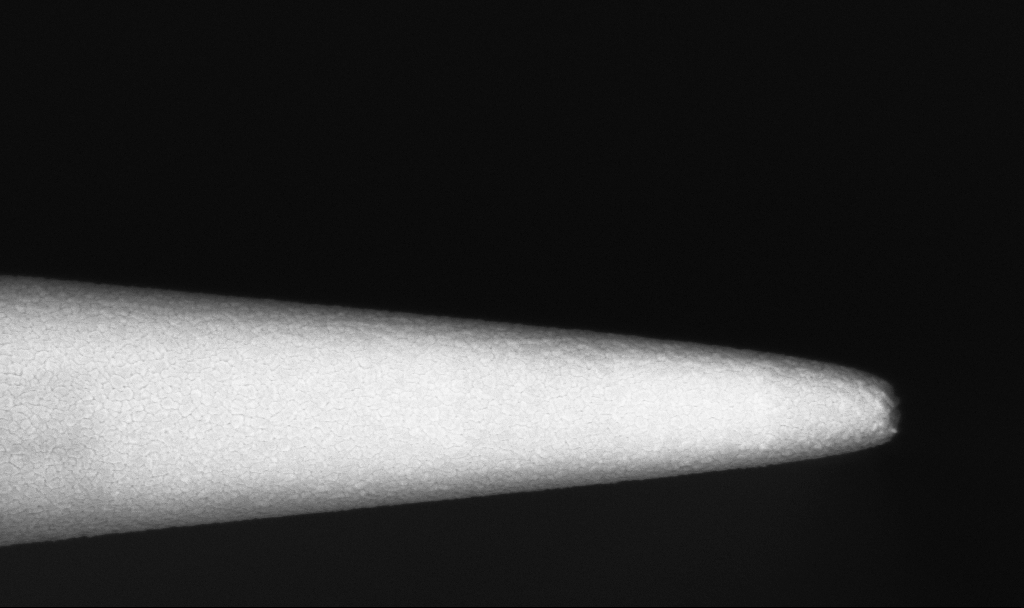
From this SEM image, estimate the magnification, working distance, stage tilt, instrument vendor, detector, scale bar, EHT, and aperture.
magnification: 47.71 K X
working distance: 3.6 mm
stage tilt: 0°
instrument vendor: Zeiss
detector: InLens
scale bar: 1000 nm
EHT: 1.5 kV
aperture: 30 µm